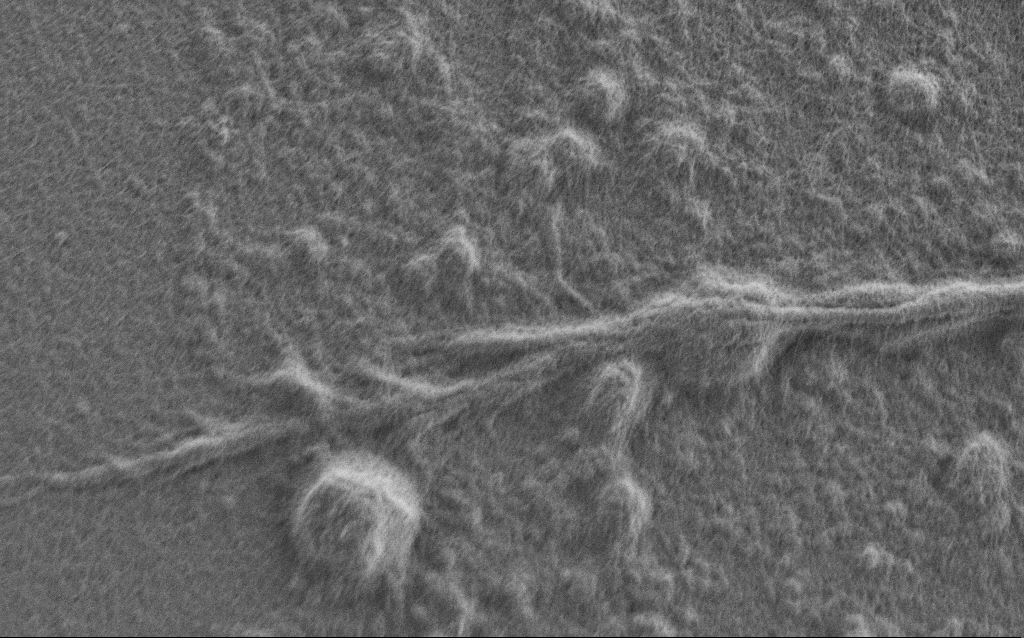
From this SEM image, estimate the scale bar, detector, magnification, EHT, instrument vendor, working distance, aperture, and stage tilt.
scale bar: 2000 nm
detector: SE2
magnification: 10 K X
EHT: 0.9 kV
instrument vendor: Zeiss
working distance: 7 mm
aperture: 30 µm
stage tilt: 0°